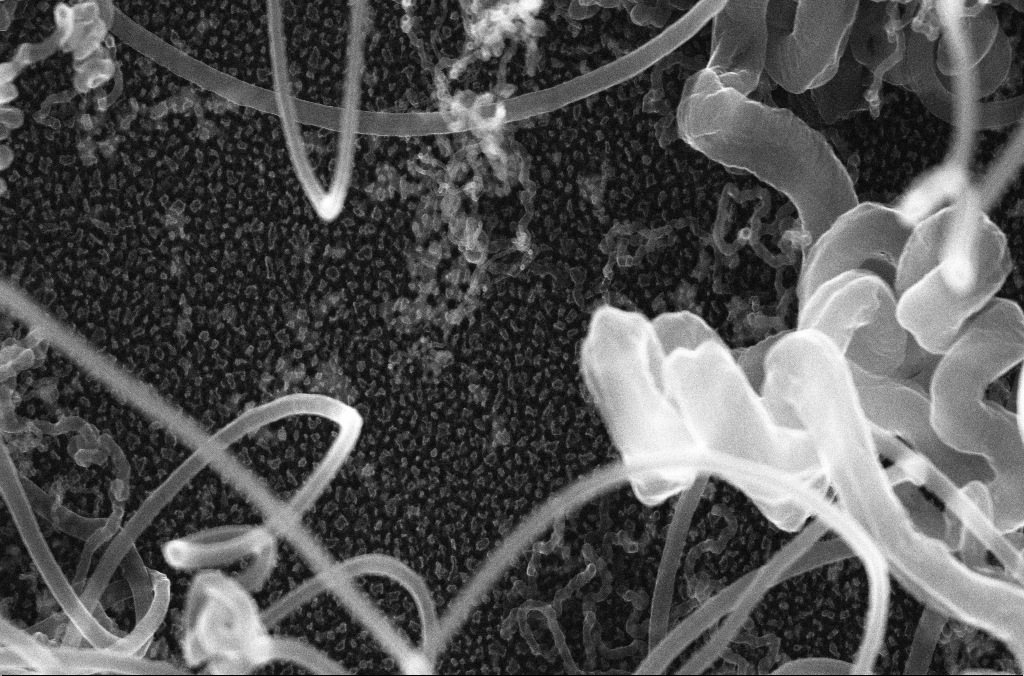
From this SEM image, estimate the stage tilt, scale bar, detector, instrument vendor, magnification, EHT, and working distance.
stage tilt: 0°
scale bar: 1000 nm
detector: InLens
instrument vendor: Zeiss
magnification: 59.09 K X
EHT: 20 kV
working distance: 4.2 mm